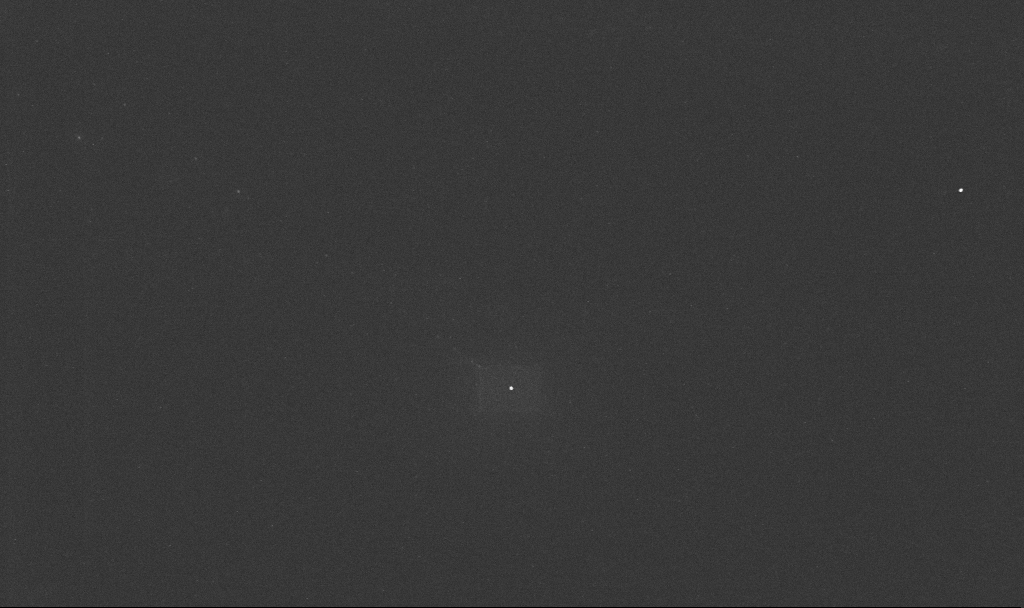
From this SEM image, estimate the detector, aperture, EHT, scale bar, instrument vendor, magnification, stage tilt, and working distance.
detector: InLens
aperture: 30 µm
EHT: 10 kV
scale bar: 1000 nm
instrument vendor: Zeiss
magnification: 59.49 K X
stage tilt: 0°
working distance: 3.2 mm